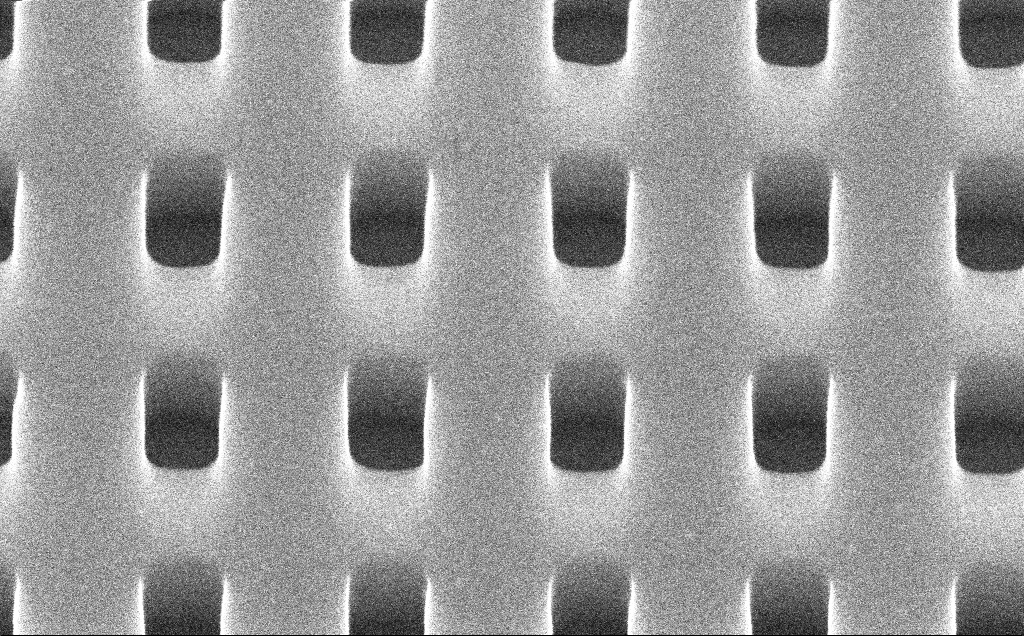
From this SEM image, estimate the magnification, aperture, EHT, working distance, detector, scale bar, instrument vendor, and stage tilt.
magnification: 150 K X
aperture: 30 µm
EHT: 10 kV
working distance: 5 mm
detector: InLens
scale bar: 200 nm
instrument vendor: Zeiss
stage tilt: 45°